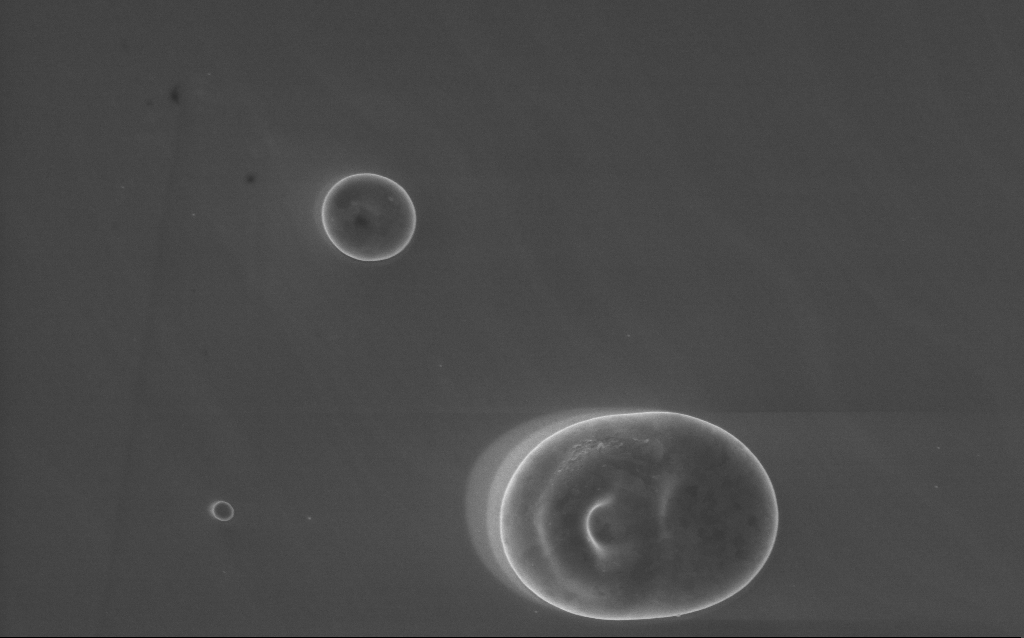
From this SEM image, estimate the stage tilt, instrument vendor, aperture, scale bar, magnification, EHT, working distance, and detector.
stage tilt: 0°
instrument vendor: Zeiss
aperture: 30 µm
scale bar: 2000 nm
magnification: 20 K X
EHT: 5 kV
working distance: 4 mm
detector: InLens